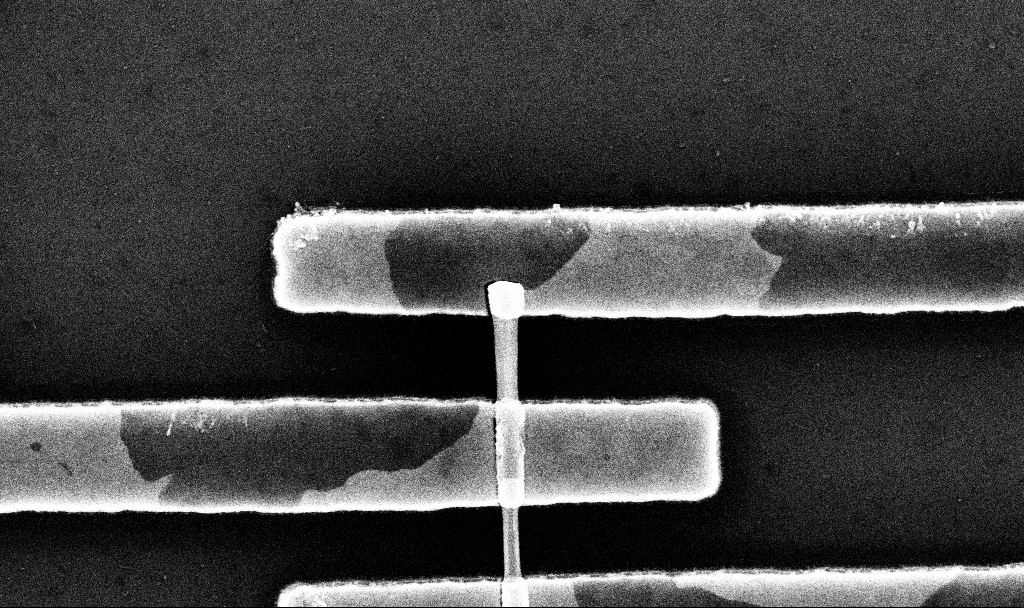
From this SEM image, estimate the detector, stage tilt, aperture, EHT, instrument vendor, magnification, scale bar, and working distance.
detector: InLens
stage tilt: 0°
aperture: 30 µm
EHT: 10 kV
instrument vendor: Zeiss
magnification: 63.69 K X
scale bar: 1000 nm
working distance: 6.8 mm